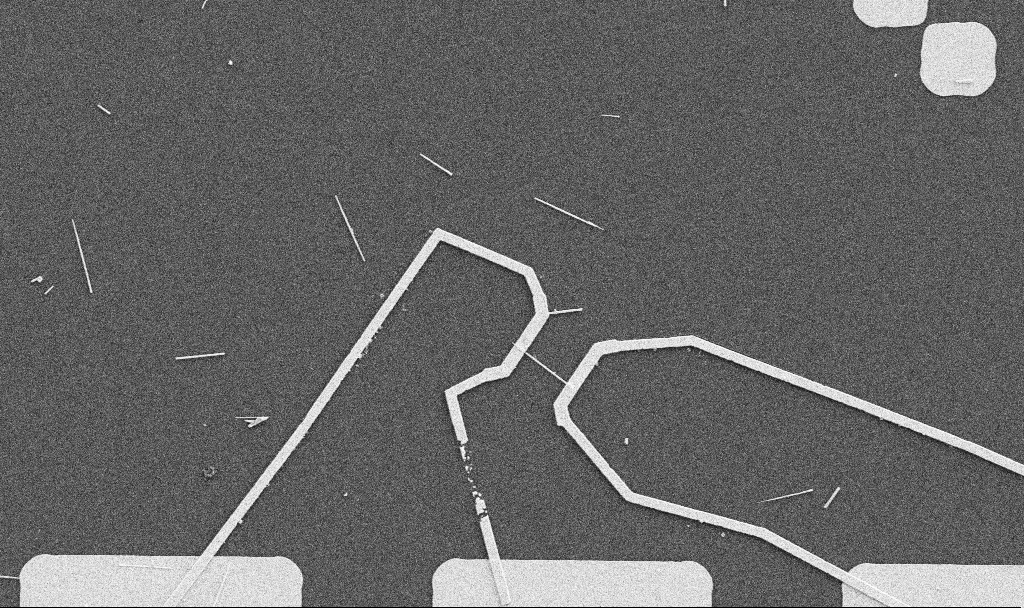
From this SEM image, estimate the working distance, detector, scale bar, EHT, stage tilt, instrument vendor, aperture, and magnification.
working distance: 10.7 mm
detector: SE2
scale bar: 10000 nm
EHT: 5 kV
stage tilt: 0°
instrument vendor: Zeiss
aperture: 30 µm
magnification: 5 K X